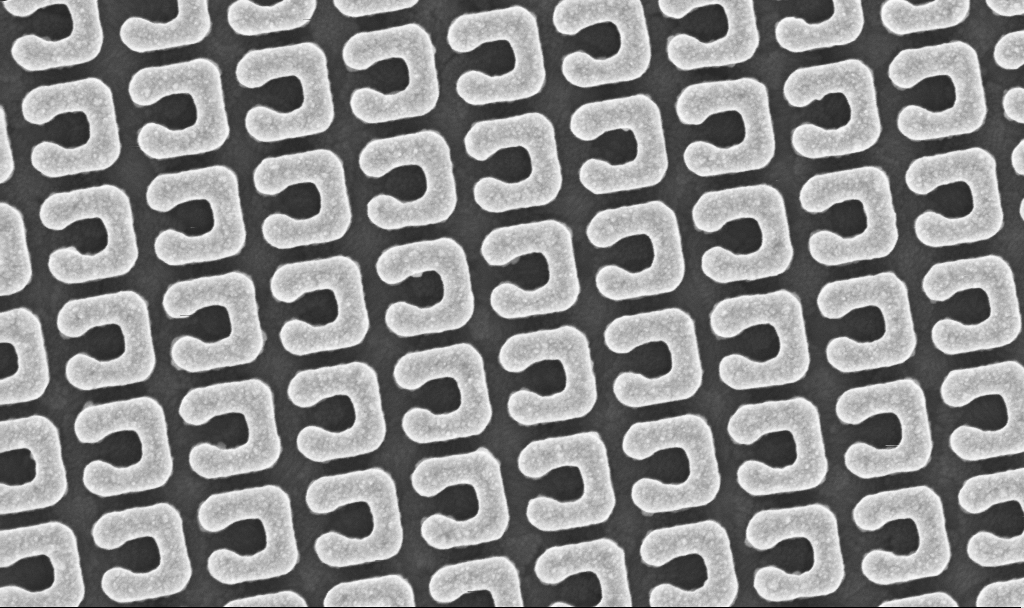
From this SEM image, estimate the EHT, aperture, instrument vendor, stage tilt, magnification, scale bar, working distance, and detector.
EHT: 5 kV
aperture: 30 µm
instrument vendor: Zeiss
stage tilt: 0°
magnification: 85.99 K X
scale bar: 200 nm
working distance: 3.9 mm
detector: InLens